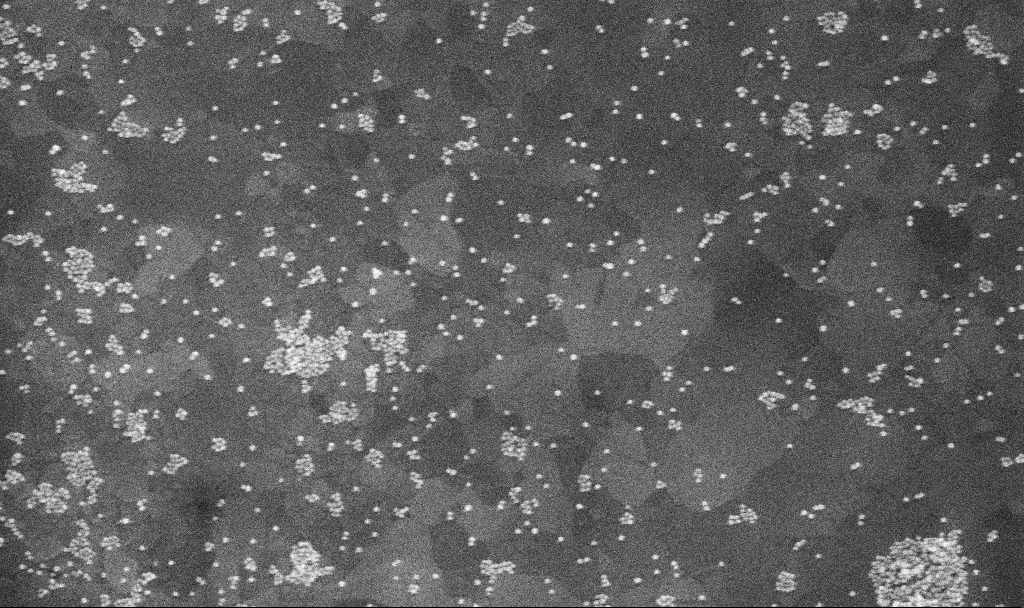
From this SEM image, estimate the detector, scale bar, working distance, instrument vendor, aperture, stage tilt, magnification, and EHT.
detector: InLens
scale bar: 200 nm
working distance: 3.8 mm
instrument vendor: Zeiss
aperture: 30 µm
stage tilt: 0°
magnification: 100 K X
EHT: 10 kV